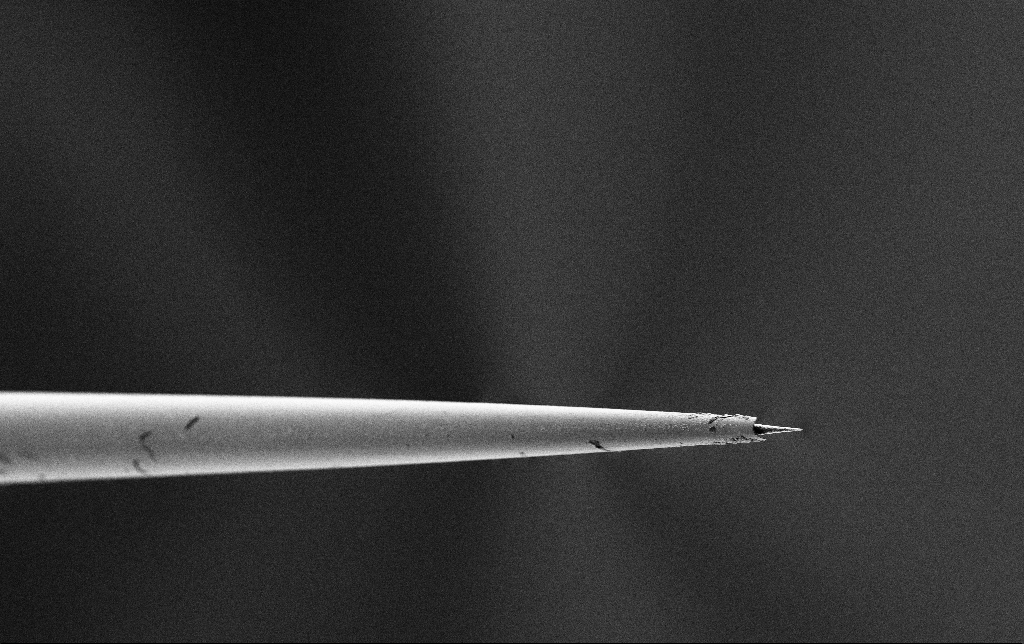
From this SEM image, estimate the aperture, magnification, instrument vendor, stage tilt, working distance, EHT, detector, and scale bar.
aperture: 30 µm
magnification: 2.5 K X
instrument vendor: Zeiss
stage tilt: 45°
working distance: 7.4 mm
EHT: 2 kV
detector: SE2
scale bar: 10000 nm